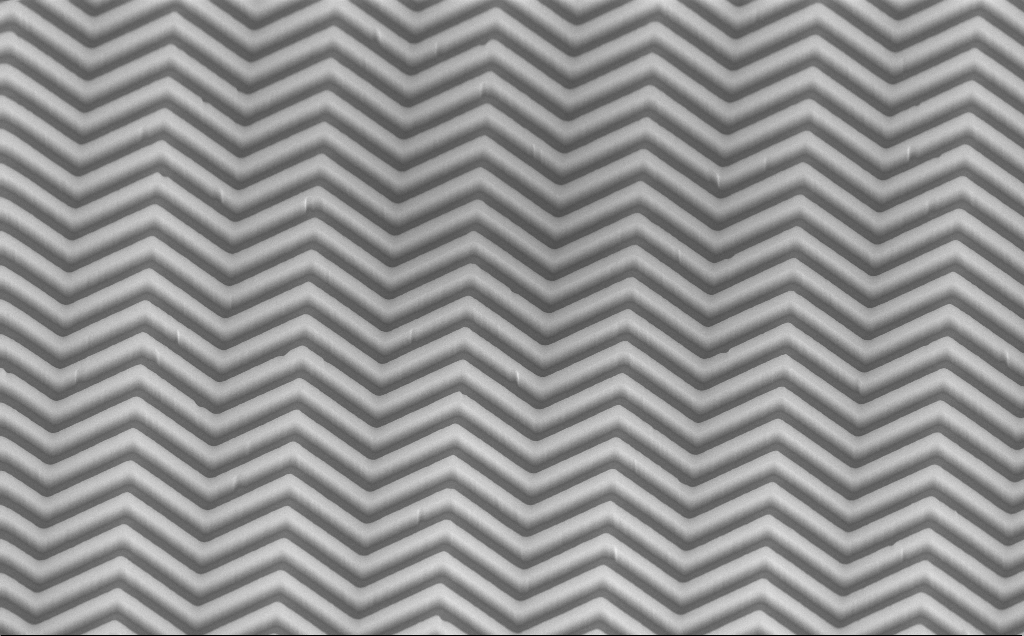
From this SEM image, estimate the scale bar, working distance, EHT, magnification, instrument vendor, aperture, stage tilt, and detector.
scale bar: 1000 nm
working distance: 6 mm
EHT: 10 kV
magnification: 29.49 K X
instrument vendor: Zeiss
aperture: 30 µm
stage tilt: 45°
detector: InLens